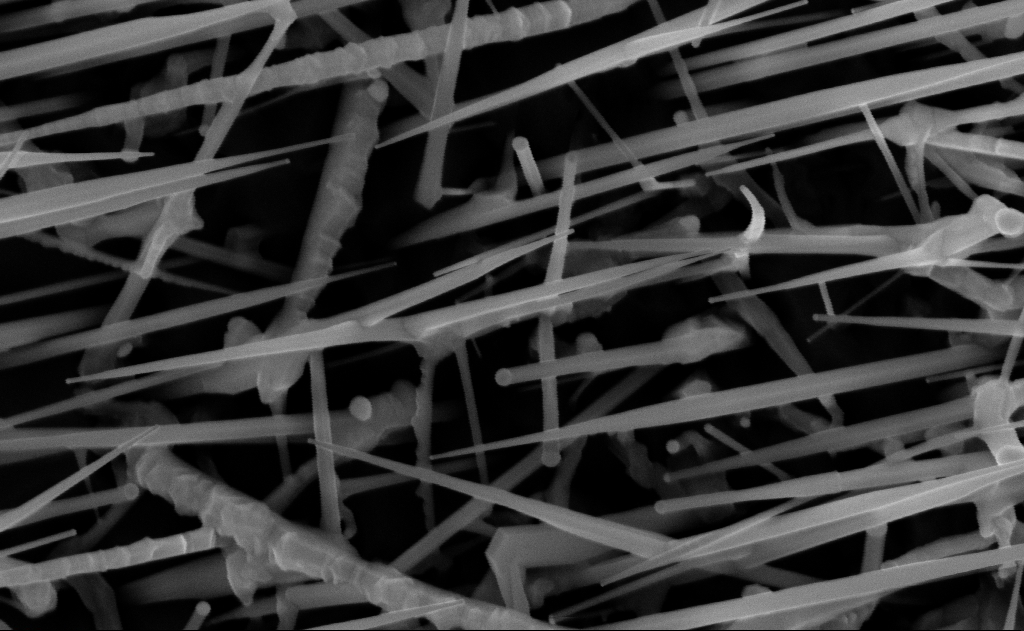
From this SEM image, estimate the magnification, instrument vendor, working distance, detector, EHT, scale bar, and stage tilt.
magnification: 80 K X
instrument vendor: Zeiss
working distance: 15 mm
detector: InLens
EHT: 10 kV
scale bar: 200 nm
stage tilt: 0°